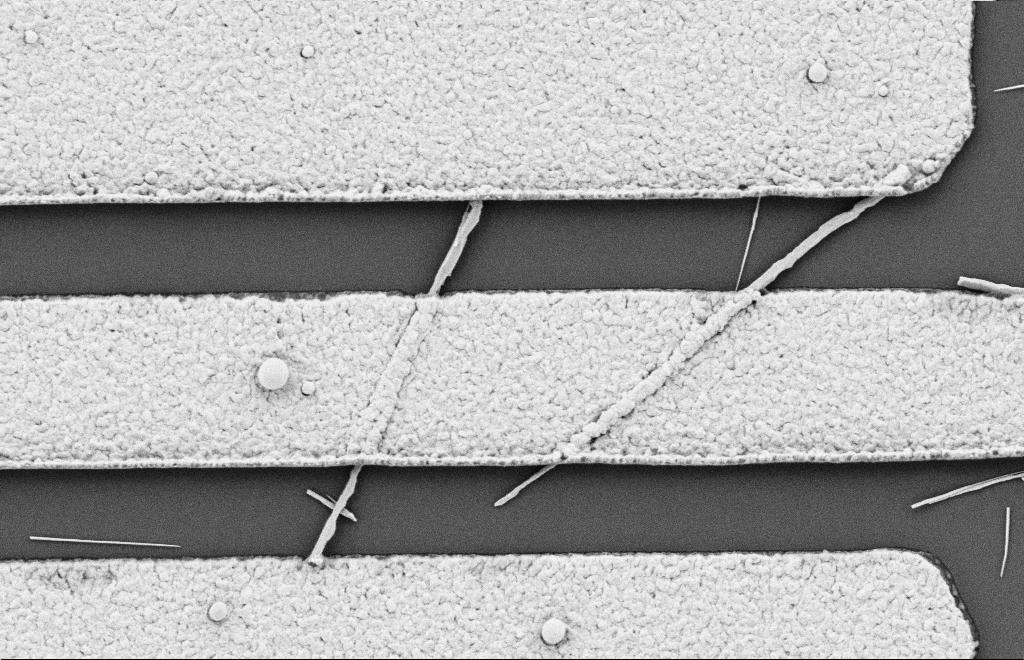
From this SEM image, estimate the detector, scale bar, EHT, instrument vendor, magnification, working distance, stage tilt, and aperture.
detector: SE2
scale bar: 1000 nm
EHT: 2 kV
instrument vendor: Zeiss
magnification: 23.87 K X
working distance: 10 mm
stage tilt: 0°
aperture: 20 µm